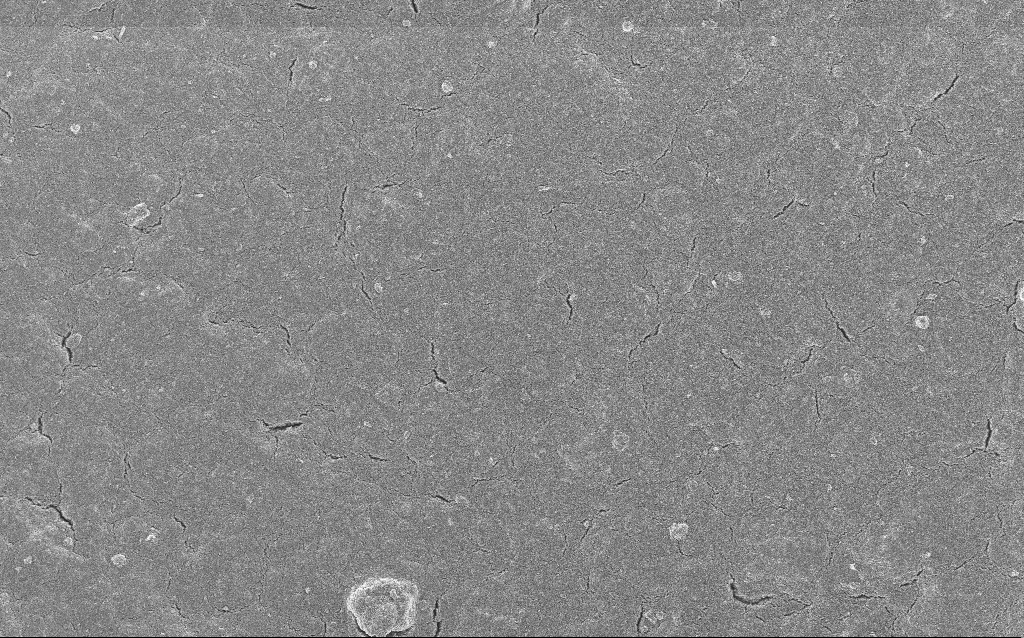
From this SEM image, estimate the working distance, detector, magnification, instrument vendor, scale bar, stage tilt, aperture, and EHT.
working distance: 4.3 mm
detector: InLens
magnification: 1.36 K X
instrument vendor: Zeiss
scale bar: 10000 nm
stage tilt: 0°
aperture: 30 µm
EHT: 5 kV